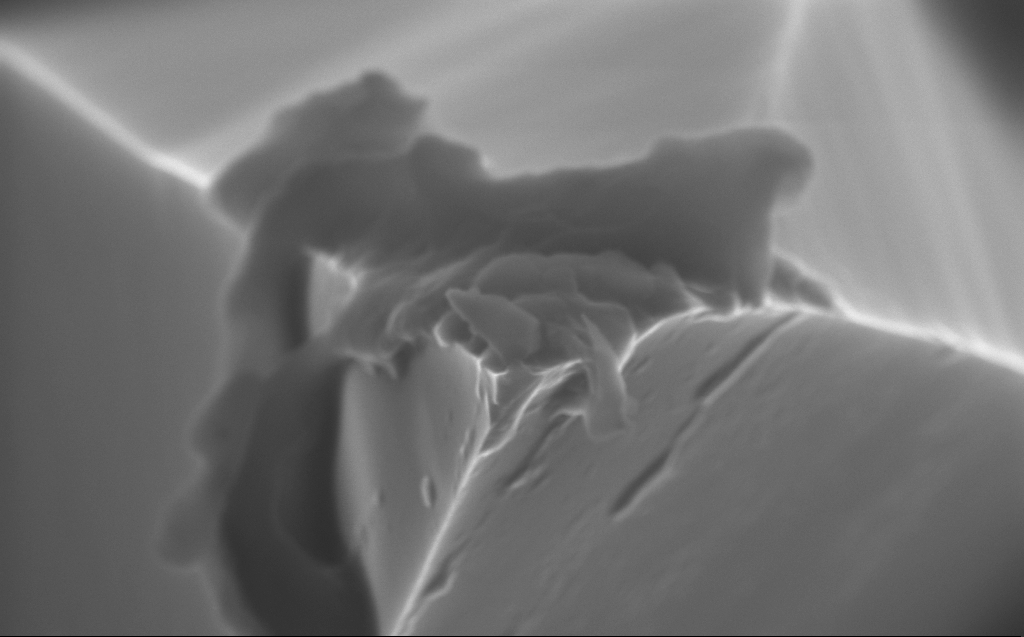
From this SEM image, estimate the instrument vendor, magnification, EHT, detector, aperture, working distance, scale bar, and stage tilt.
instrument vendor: Zeiss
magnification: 62.67 K X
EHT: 10 kV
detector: InLens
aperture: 30 µm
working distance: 4 mm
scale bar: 1000 nm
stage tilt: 0°